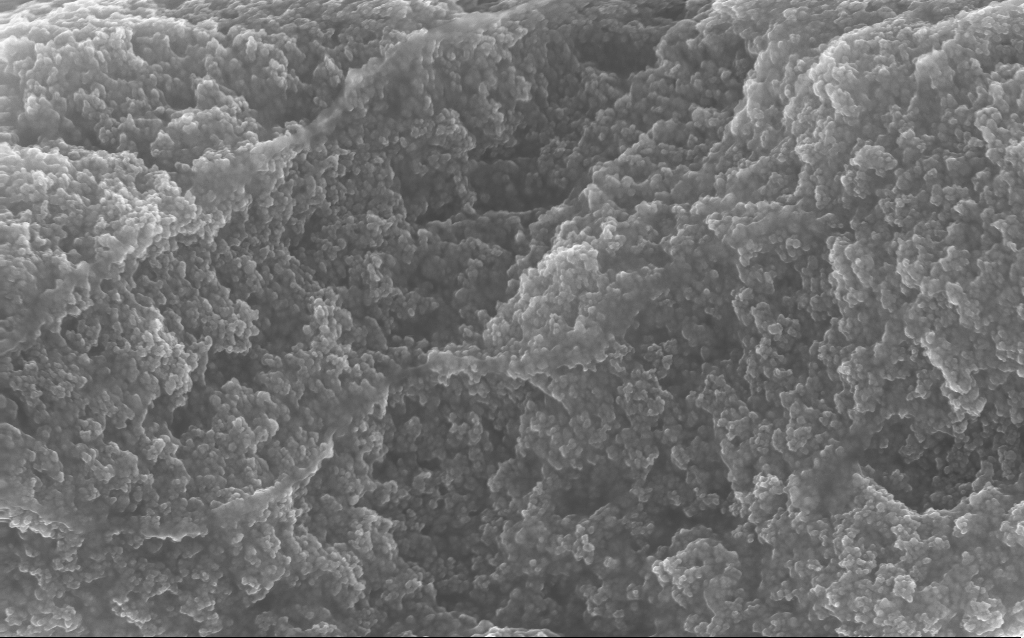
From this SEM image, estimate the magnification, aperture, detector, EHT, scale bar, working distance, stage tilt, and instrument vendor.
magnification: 65.04 K X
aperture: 30 µm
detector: InLens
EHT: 10 kV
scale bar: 1000 nm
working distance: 2.8 mm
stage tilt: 0°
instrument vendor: Zeiss